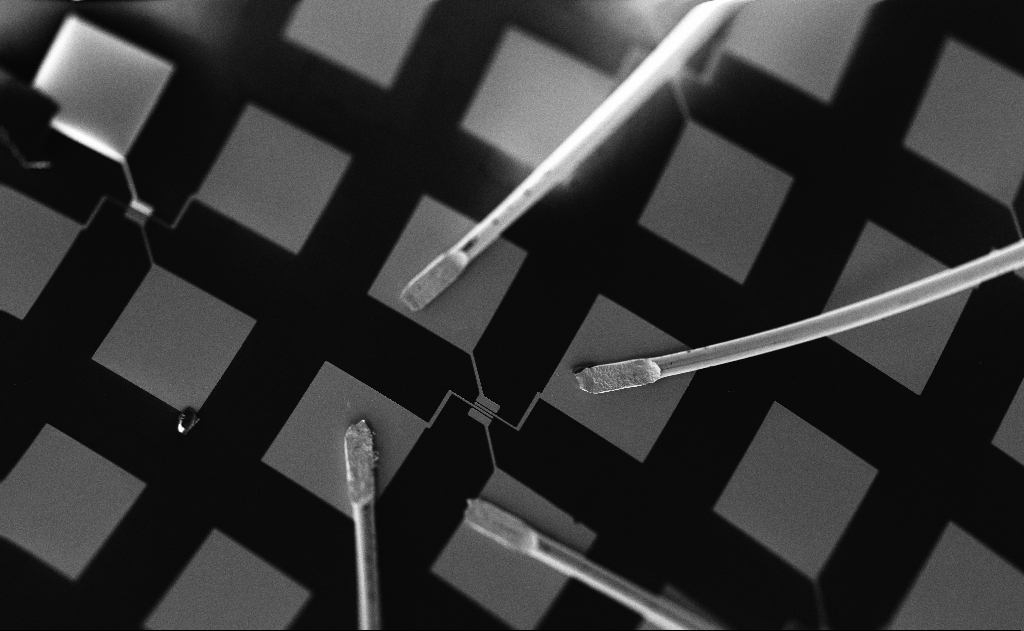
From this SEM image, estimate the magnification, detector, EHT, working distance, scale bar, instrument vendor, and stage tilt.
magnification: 0.297 K X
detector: InLens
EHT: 5 kV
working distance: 21 mm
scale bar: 100000 nm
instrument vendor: Zeiss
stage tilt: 0°